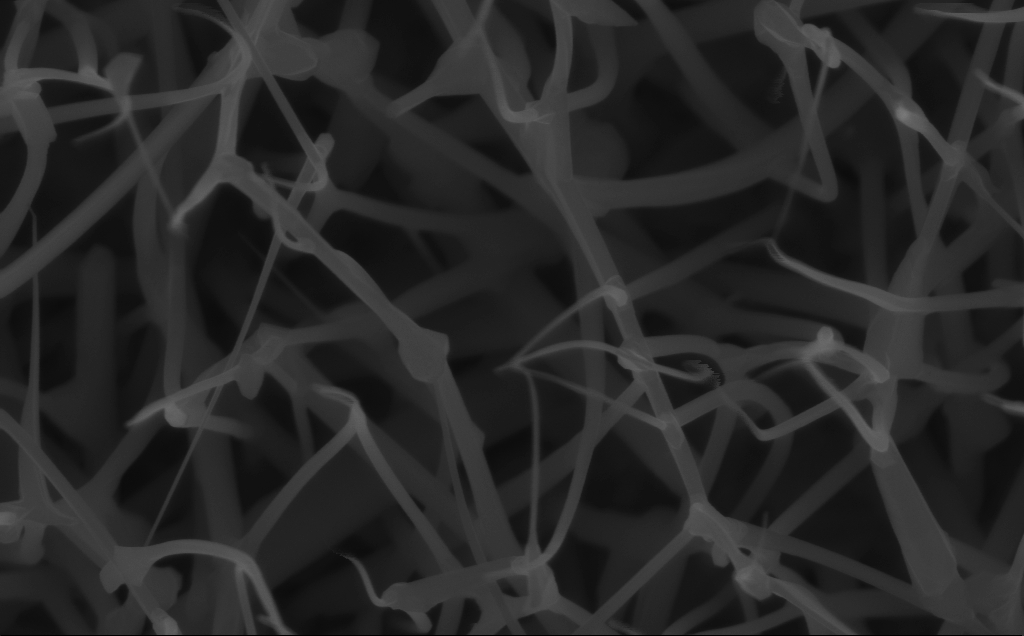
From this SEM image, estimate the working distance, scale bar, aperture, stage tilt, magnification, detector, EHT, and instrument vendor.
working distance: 6 mm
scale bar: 200 nm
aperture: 30 µm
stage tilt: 0°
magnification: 80 K X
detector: InLens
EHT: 10 kV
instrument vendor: Zeiss